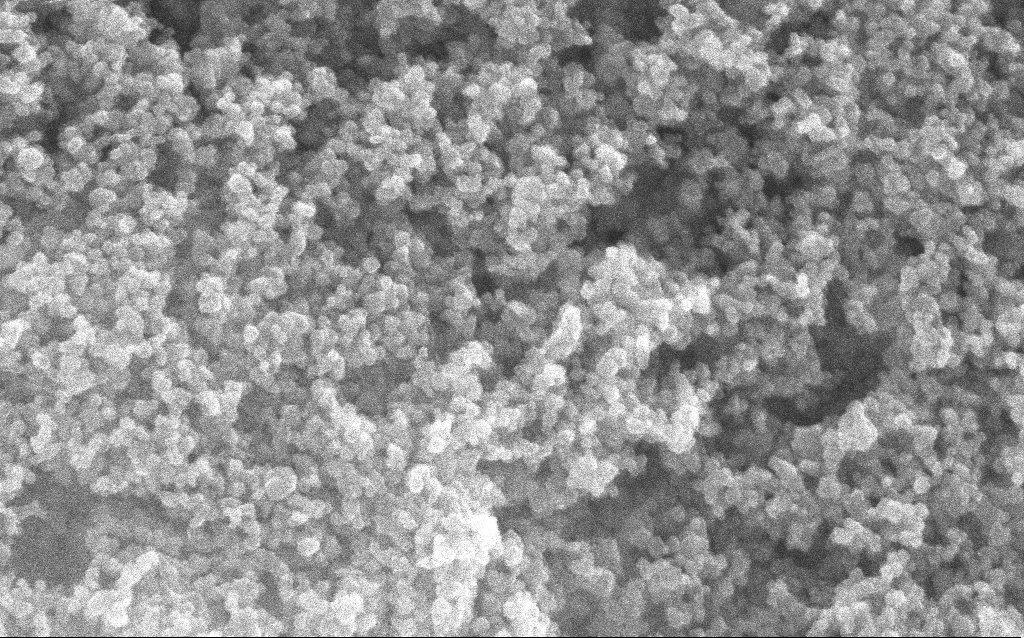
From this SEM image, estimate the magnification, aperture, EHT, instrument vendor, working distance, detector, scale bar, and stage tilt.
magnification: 204.13 K X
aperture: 30 µm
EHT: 10 kV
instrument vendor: Zeiss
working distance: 2.4 mm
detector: InLens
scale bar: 100 nm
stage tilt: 0°